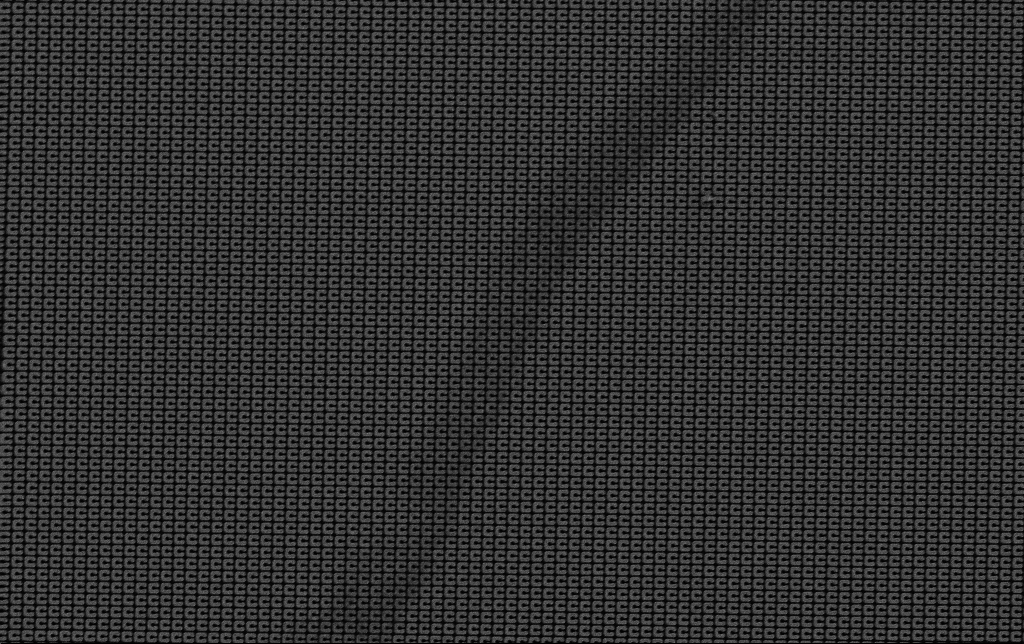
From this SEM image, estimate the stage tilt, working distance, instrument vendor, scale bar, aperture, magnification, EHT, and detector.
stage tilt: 0°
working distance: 5.1 mm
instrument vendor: Zeiss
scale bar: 2000 nm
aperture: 30 µm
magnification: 9.86 K X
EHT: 3 kV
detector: SE2